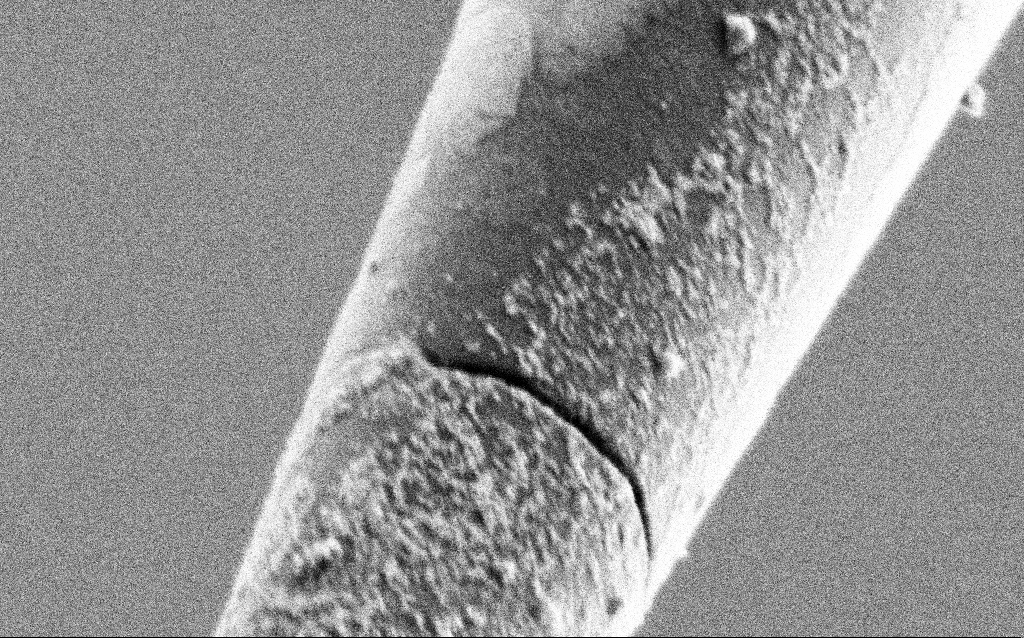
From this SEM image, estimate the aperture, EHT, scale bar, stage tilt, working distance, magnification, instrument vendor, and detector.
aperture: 30 µm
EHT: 1 kV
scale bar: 200 nm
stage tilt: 45°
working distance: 6.7 mm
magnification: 100 K X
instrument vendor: Zeiss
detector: SE2